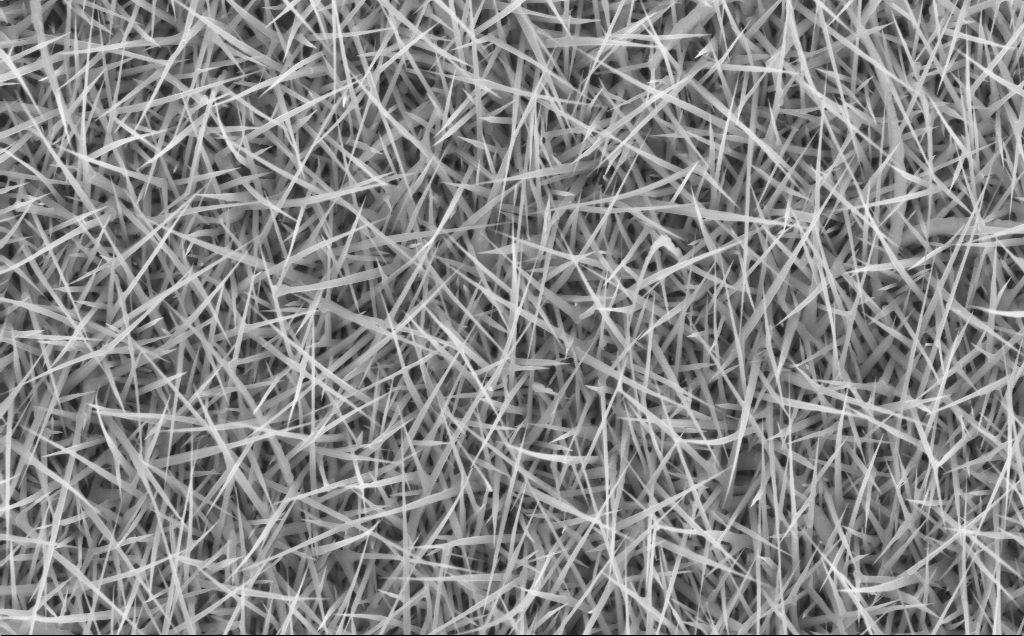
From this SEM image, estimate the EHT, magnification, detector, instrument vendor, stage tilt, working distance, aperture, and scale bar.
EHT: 10 kV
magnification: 20 K X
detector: InLens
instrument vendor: Zeiss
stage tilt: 0°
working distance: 7 mm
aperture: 30 µm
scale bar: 2000 nm